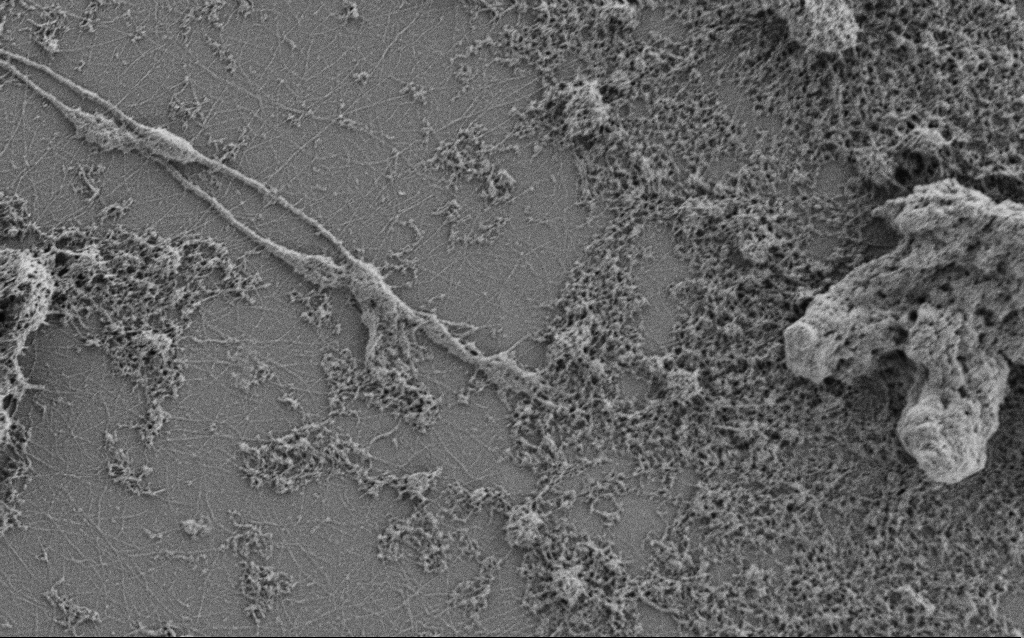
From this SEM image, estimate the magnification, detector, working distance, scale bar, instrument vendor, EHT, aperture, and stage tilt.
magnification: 10 K X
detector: SE2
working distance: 4 mm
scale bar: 2000 nm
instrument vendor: Zeiss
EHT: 0.9 kV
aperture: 30 µm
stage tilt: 0°